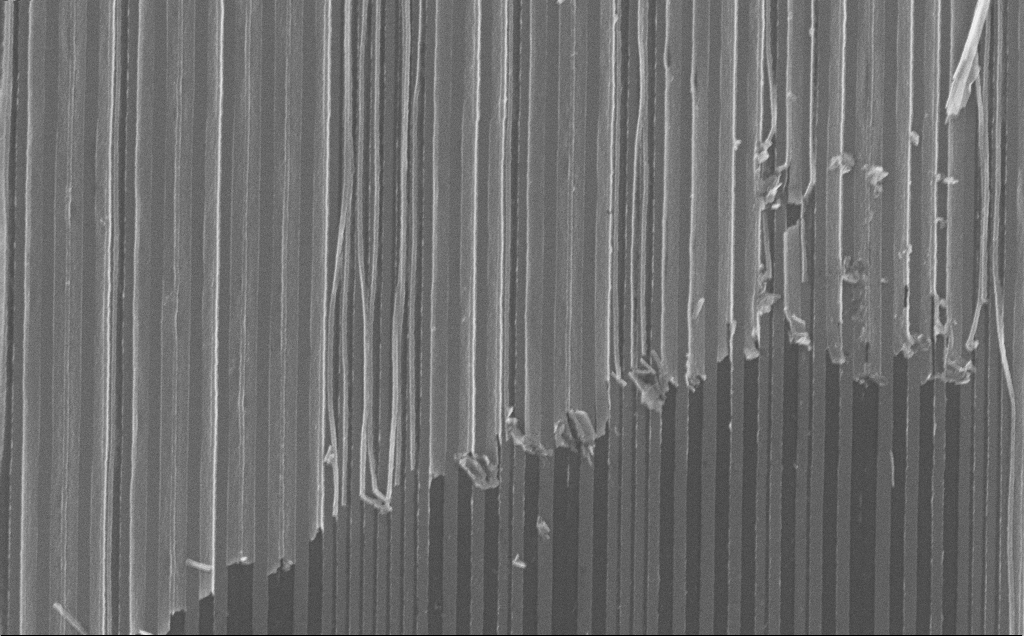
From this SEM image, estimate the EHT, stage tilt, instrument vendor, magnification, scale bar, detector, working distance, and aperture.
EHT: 10 kV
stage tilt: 0°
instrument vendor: Zeiss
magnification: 24.95 K X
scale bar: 2000 nm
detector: InLens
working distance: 7 mm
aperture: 30 µm